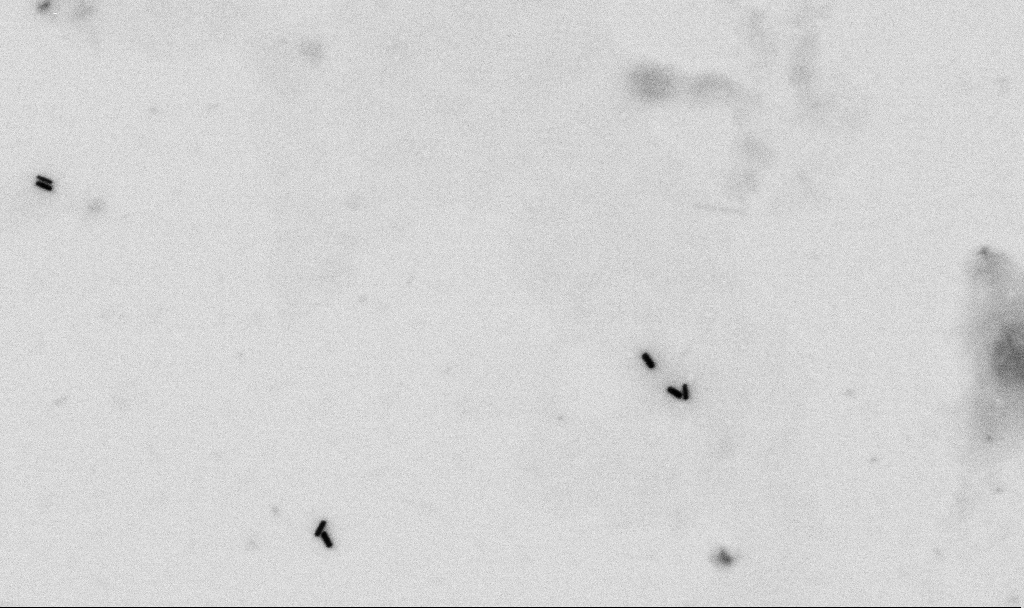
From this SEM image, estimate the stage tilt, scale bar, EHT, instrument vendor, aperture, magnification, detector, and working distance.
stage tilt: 0°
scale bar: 200 nm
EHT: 2 kV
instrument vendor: Zeiss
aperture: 30 µm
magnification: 80 K X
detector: SE2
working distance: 4.5 mm